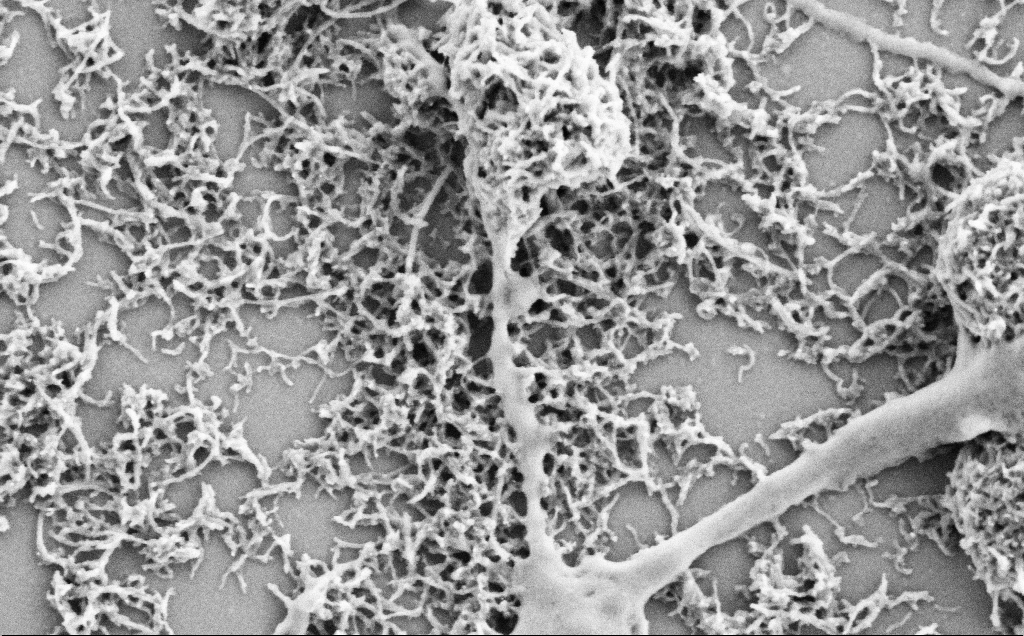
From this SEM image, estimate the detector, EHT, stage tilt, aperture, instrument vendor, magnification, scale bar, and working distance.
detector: SE2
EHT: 2 kV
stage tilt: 0°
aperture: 30 µm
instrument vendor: Zeiss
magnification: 50 K X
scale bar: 1000 nm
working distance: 7.1 mm